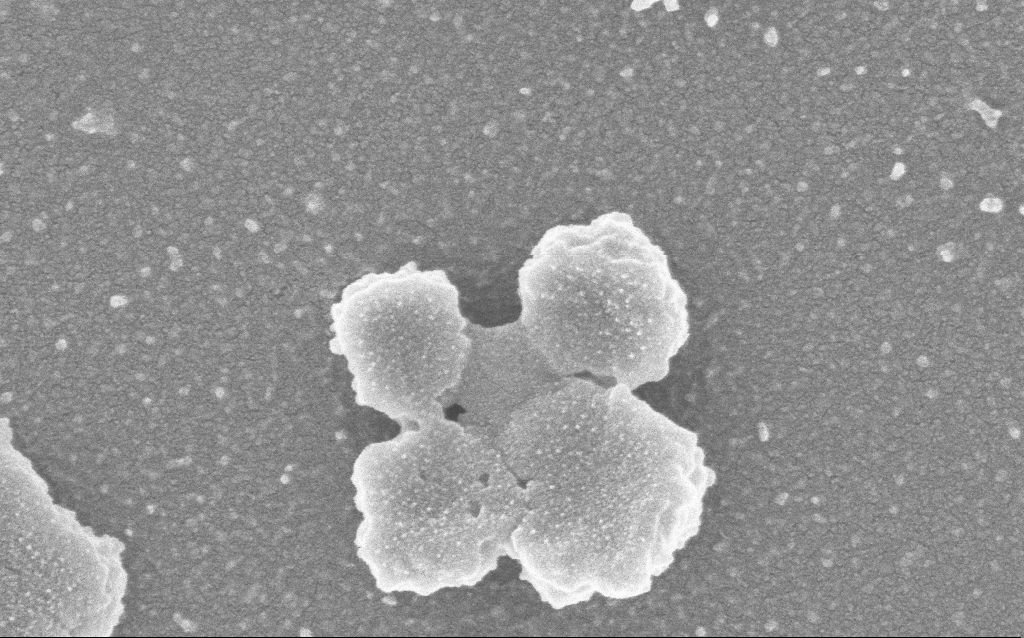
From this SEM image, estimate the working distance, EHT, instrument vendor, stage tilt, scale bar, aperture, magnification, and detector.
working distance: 2.5 mm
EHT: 3 kV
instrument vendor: Zeiss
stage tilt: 0°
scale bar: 200 nm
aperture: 30 µm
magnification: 150 K X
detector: InLens